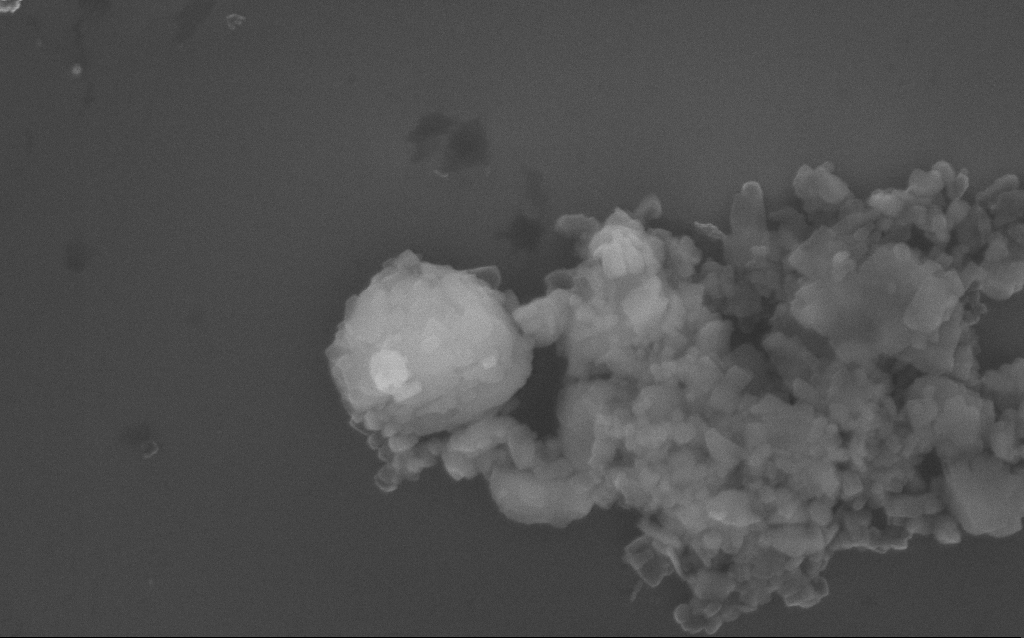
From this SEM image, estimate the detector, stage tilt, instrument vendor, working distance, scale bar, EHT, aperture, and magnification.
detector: InLens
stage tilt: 0°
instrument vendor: Zeiss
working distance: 3 mm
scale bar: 1000 nm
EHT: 10 kV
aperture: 30 µm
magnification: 70.45 K X